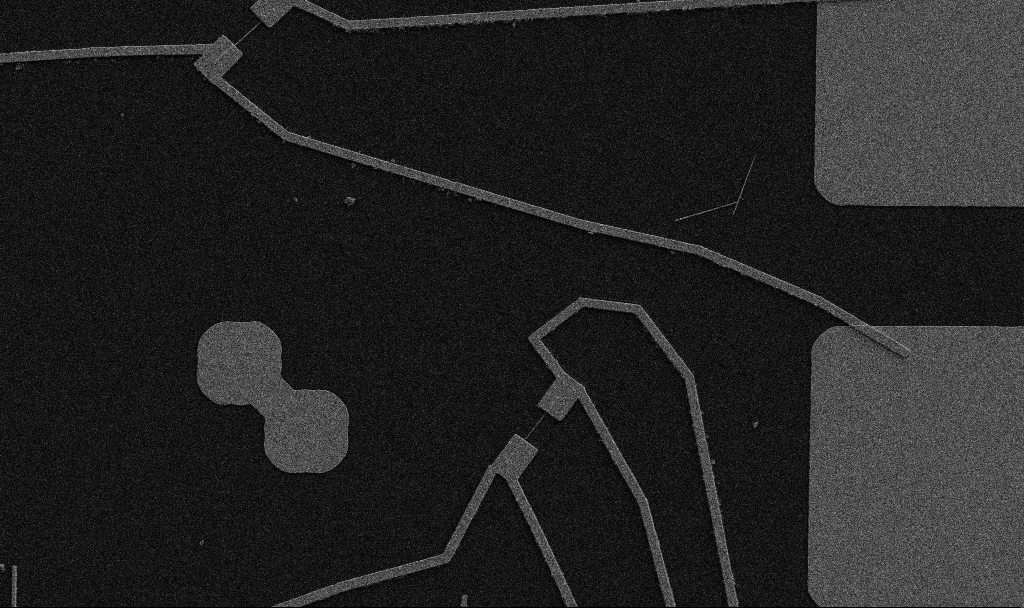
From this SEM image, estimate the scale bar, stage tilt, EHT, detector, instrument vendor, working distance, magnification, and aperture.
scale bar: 10000 nm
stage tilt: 0°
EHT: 5 kV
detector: SE2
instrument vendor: Zeiss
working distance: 10.7 mm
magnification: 5 K X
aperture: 30 µm